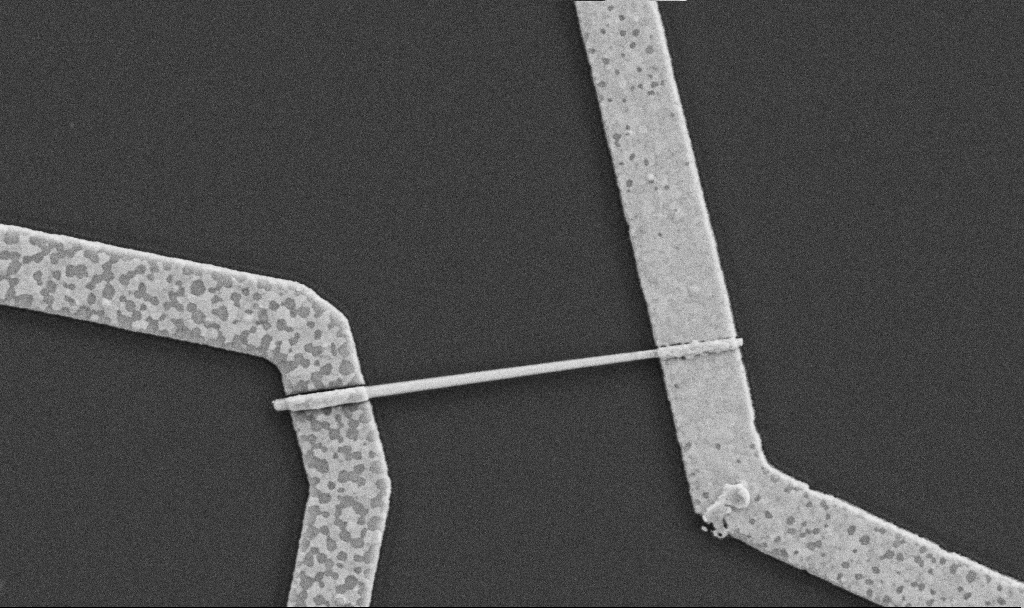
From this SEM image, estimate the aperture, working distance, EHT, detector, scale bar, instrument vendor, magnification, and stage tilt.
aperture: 30 µm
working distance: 8.7 mm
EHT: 5 kV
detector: SE2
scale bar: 1000 nm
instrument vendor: Zeiss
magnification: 30 K X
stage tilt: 0°